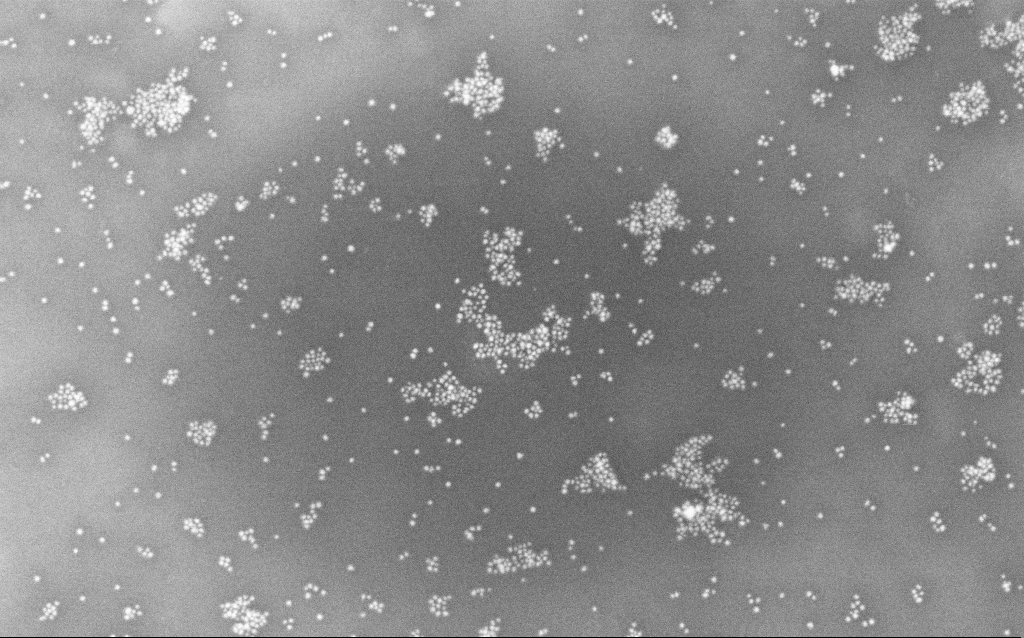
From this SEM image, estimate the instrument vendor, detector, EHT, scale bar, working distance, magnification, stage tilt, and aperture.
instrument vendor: Zeiss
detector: InLens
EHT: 10 kV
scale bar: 200 nm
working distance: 1.8 mm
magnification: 100 K X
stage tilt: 0°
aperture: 30 µm